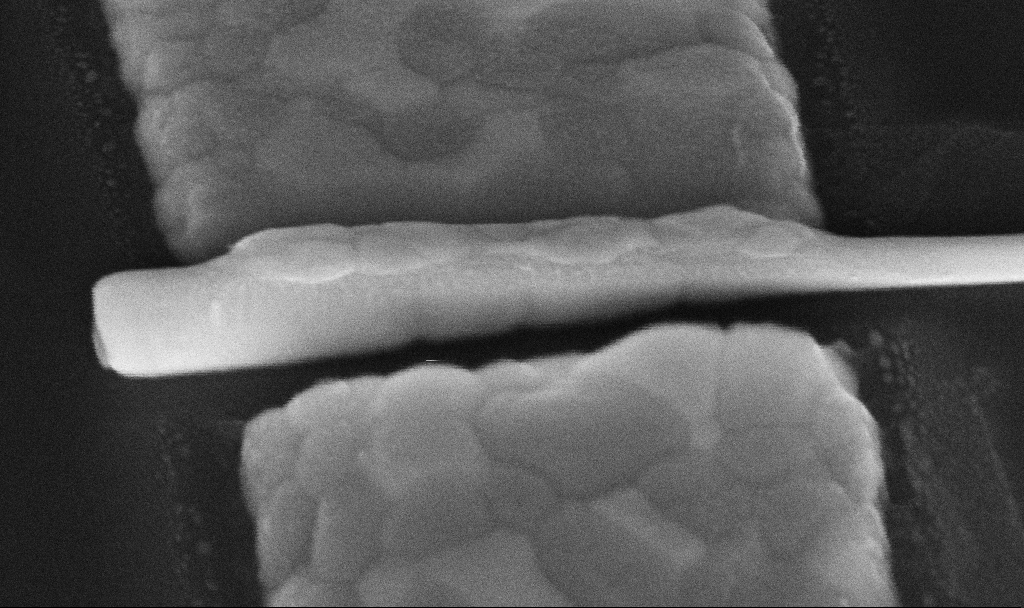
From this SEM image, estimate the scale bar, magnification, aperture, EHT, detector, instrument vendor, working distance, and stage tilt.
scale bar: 200 nm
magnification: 330.4 K X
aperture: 30 µm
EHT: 10 kV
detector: InLens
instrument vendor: Zeiss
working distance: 8.6 mm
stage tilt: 45°